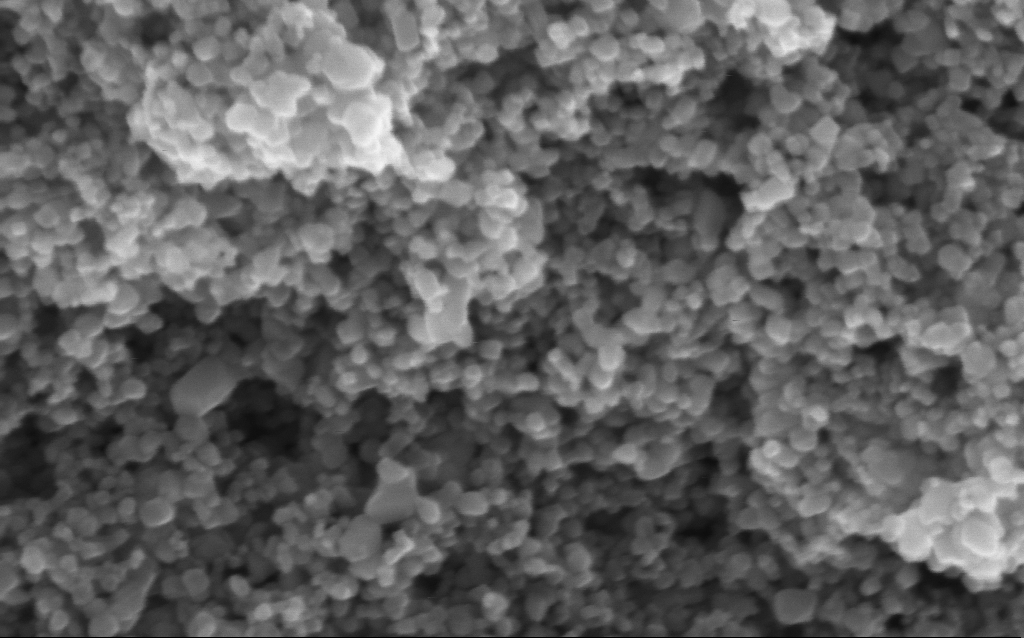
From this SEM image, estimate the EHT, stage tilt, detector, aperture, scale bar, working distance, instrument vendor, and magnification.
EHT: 5 kV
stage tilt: -0°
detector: InLens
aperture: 30 µm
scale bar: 200 nm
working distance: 4 mm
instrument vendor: Zeiss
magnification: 282.06 K X